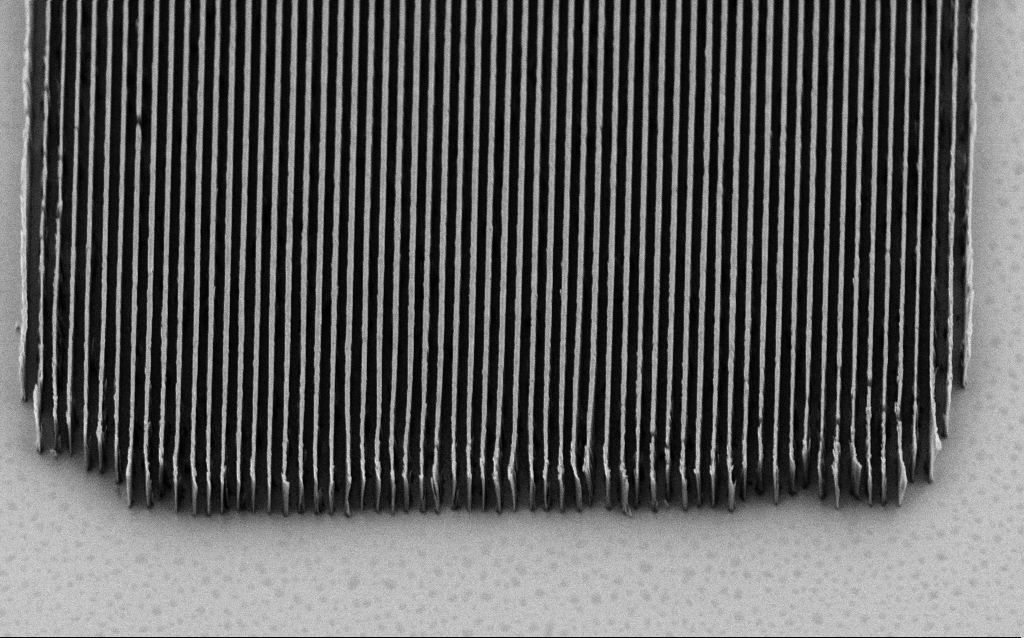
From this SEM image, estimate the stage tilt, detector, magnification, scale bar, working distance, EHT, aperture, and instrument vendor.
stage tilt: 45°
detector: SE2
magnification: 13.96 K X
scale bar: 1000 nm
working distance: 7 mm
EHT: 2 kV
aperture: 30 µm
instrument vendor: Zeiss